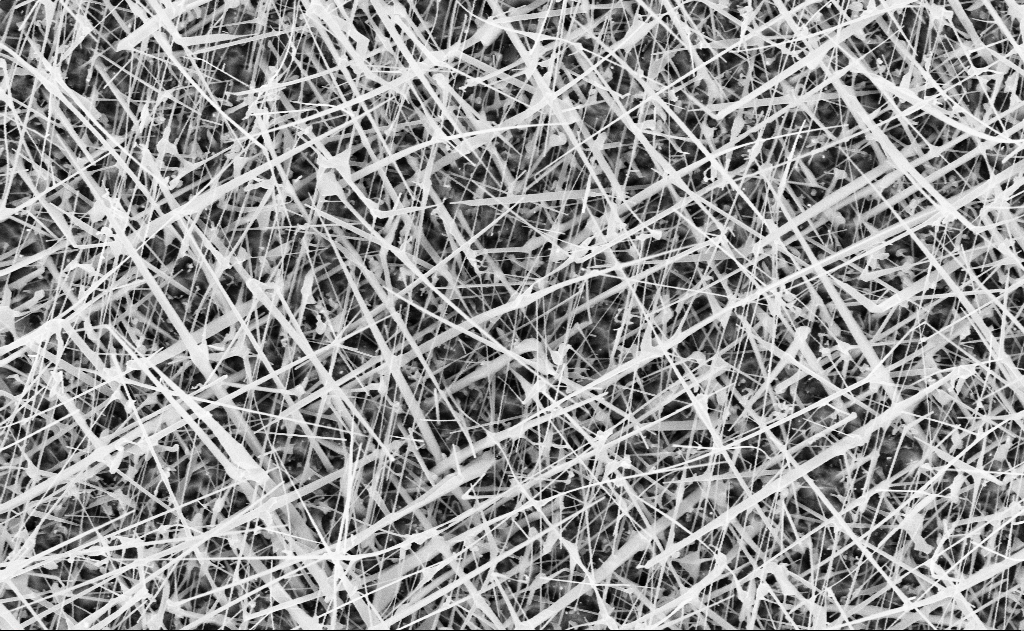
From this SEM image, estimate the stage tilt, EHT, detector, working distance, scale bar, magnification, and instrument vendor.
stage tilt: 0°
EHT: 10 kV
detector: InLens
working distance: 20 mm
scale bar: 2000 nm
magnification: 20 K X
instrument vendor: Zeiss